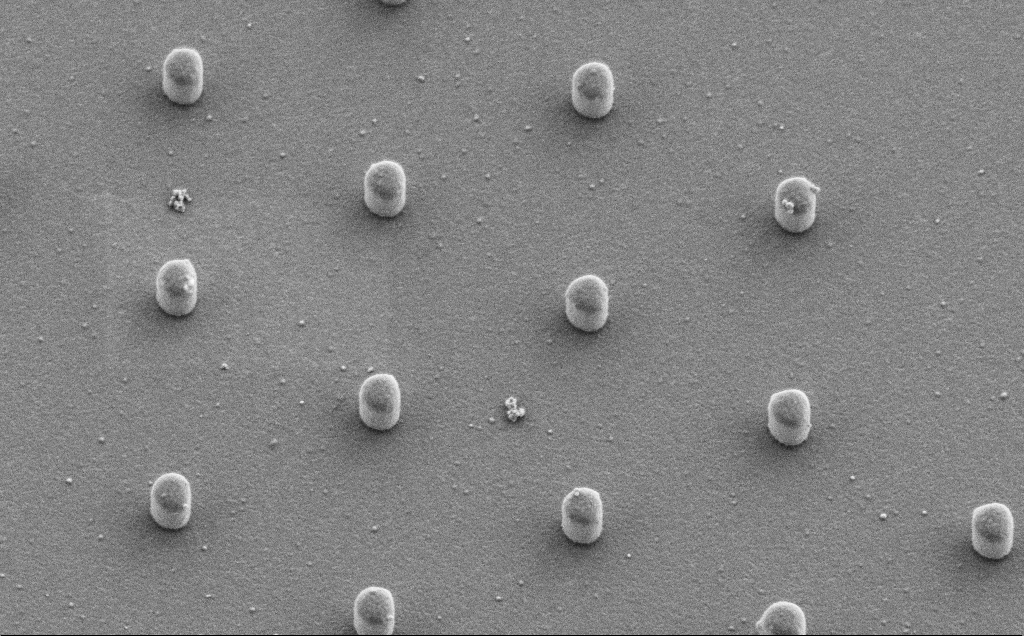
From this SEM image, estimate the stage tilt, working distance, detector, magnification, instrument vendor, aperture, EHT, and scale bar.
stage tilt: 23.9°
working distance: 7 mm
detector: SE2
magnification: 13.45 K X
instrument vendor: Zeiss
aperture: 30 µm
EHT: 1.5 kV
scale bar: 2000 nm